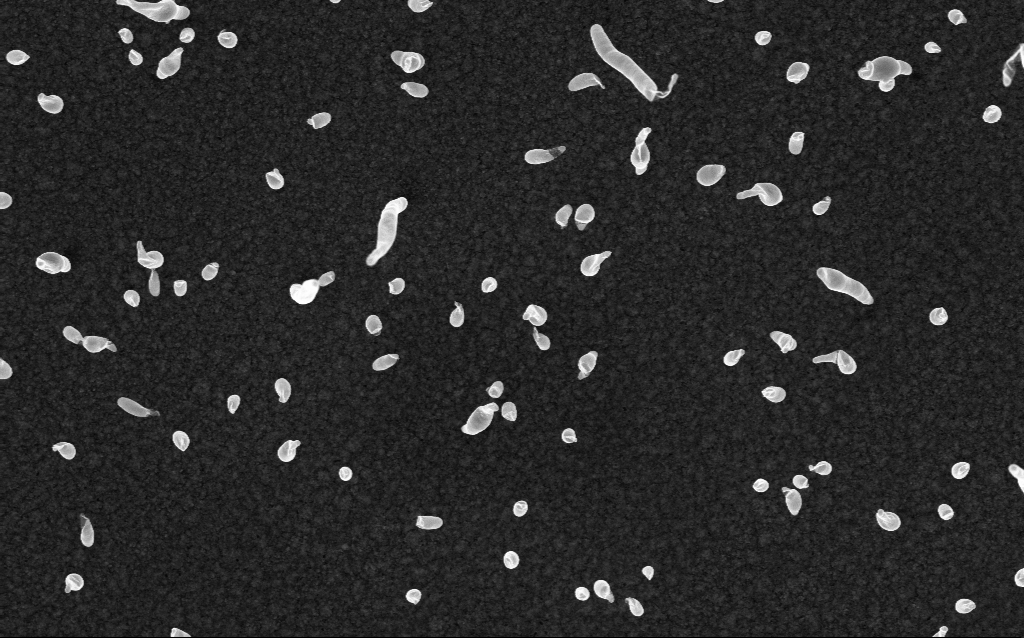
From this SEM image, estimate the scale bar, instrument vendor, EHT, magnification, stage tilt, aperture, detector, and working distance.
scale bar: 1000 nm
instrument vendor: Zeiss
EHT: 5 kV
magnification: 50 K X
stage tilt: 0°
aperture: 30 µm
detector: InLens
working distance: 2.1 mm